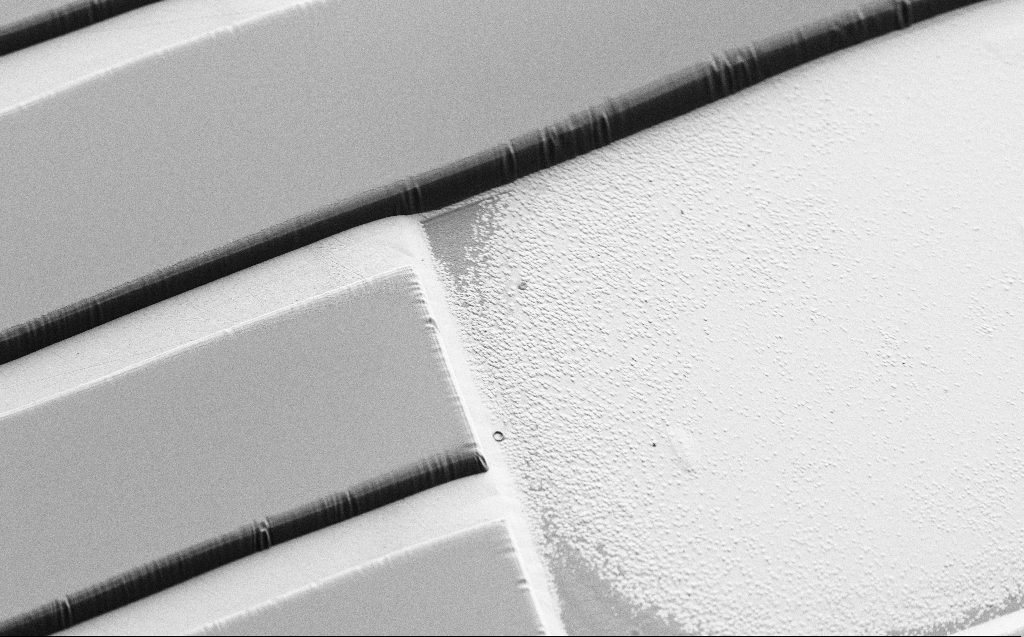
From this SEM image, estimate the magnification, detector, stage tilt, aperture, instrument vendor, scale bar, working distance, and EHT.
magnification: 0.955 K X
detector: SE2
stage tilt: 45°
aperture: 30 µm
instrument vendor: Zeiss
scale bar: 20000 nm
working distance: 4 mm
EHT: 1 kV